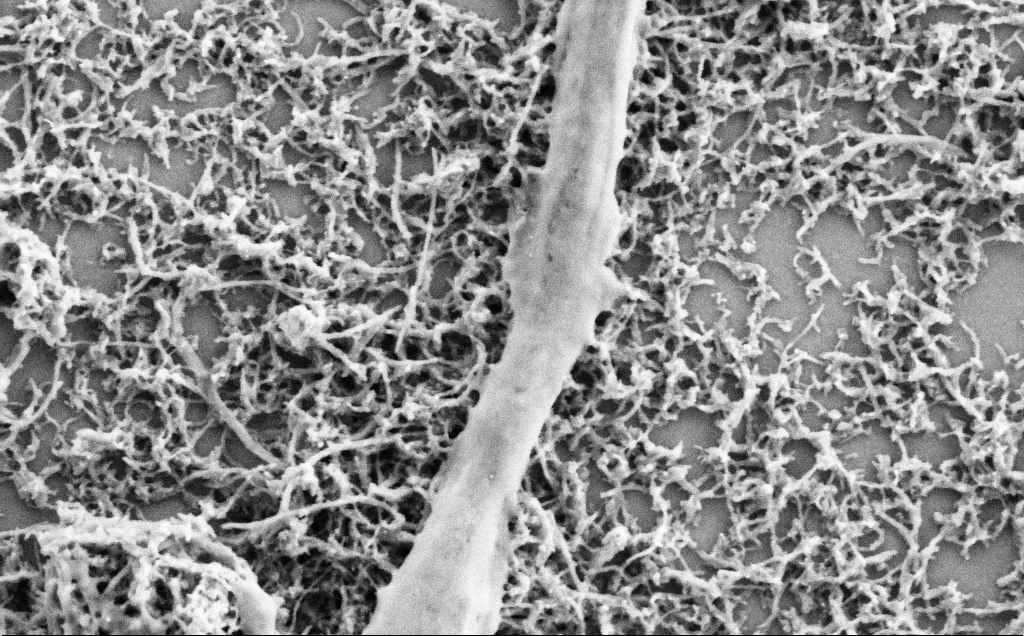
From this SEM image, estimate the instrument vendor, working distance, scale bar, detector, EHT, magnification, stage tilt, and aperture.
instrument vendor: Zeiss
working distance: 7.1 mm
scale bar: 1000 nm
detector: SE2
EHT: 2 kV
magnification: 50 K X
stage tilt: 0°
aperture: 30 µm